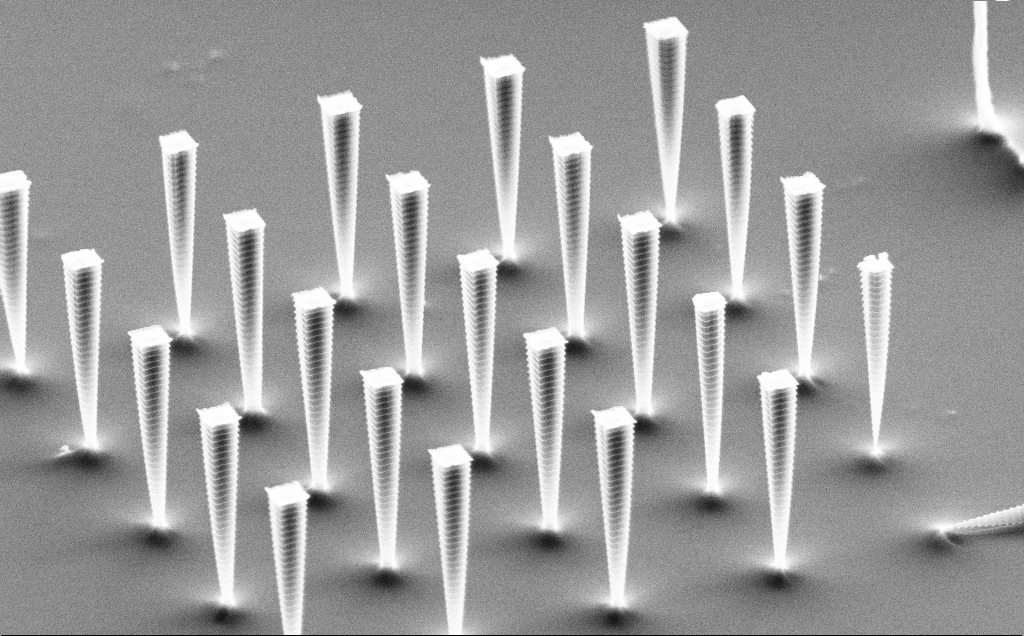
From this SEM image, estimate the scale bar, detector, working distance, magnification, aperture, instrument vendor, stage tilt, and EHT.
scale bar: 10000 nm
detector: SE2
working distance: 9 mm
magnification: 6.54 K X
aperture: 30 µm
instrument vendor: Zeiss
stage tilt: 61.4°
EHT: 10 kV